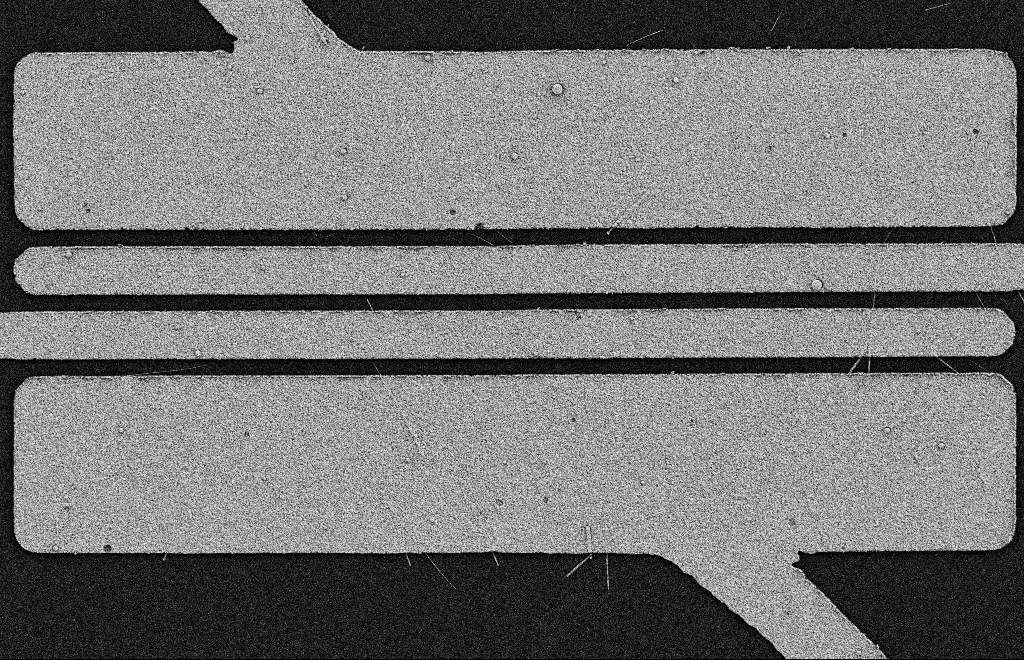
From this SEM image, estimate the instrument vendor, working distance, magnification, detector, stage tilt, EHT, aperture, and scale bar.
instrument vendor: Zeiss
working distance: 11 mm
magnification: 5.94 K X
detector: SE2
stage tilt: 0°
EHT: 2 kV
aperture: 20 µm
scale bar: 2000 nm